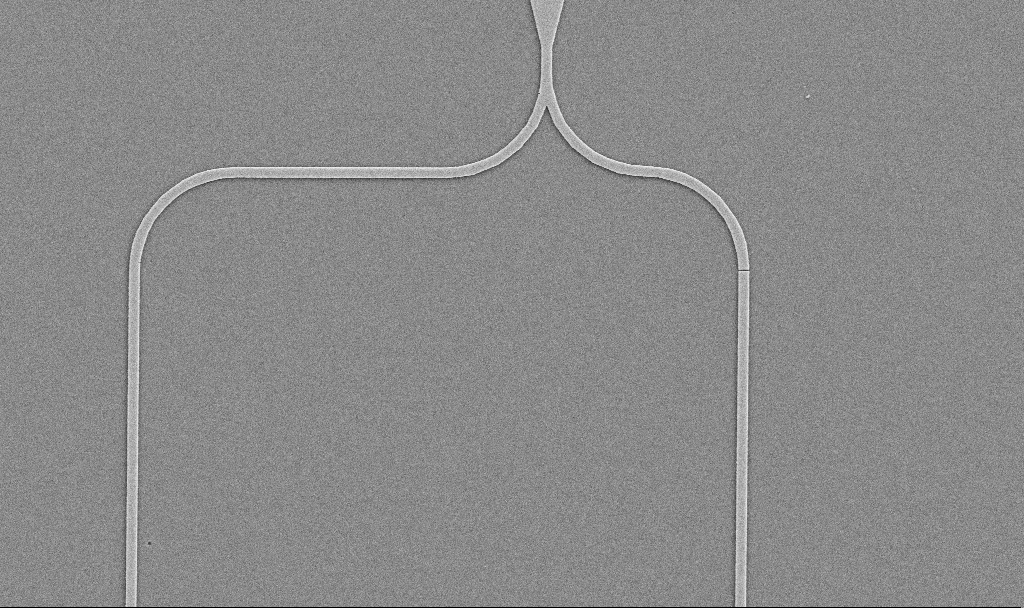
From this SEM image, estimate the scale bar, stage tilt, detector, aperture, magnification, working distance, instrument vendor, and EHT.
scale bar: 2000 nm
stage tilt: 0°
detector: SE2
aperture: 30 µm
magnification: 7.7 K X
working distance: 10.3 mm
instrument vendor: Zeiss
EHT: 5 kV